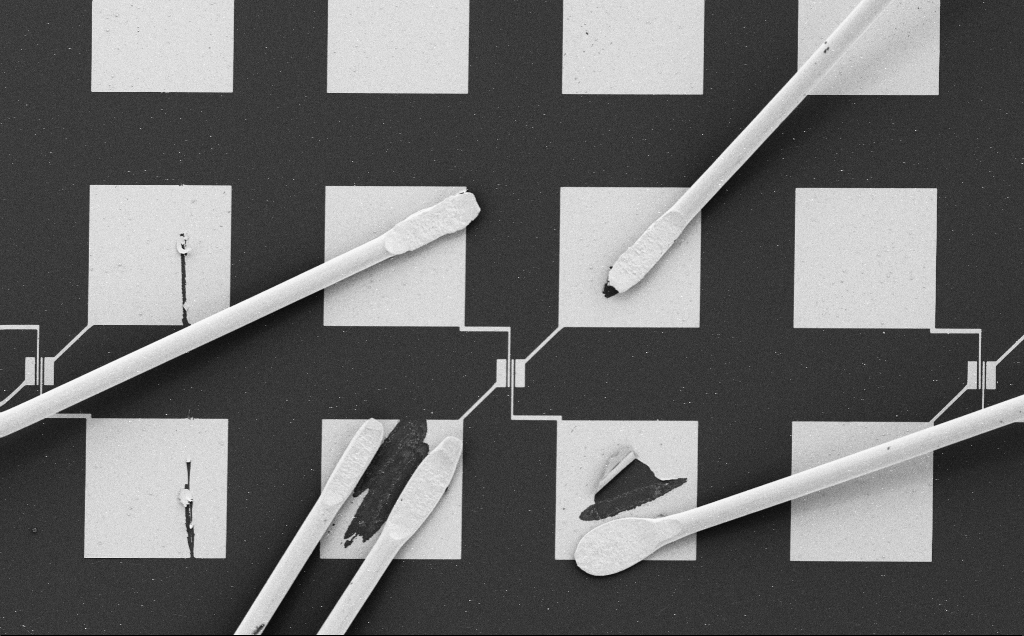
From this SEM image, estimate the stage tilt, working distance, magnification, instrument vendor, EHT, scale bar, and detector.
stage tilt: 0°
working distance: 10 mm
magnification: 0.346 K X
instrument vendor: Zeiss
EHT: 5 kV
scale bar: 200000 nm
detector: SE2